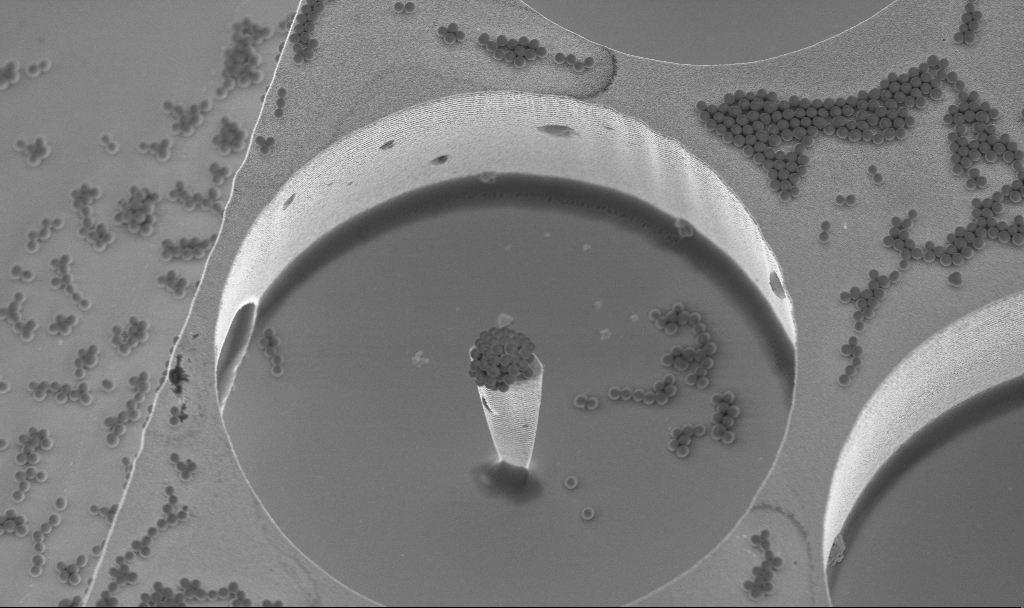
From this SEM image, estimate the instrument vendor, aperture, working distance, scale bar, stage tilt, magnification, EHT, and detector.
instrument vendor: Zeiss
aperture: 30 µm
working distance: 3.1 mm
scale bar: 10000 nm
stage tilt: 20°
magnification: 7.15 K X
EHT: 3 kV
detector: InLens